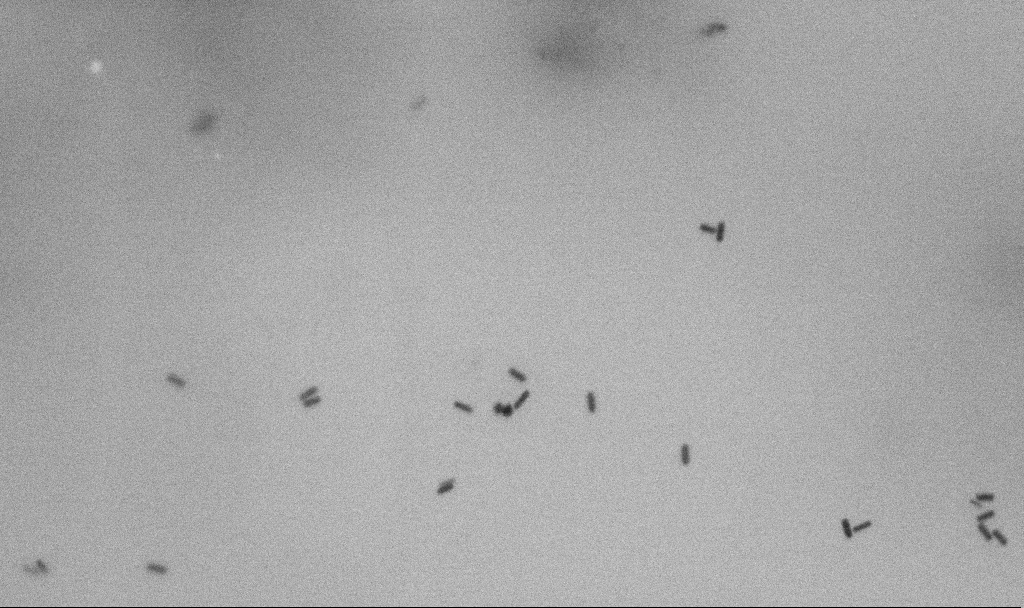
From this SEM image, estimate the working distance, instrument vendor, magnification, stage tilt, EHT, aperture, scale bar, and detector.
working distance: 4.7 mm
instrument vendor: Zeiss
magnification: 100 K X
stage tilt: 0°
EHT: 5 kV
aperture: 30 µm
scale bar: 200 nm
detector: SE2